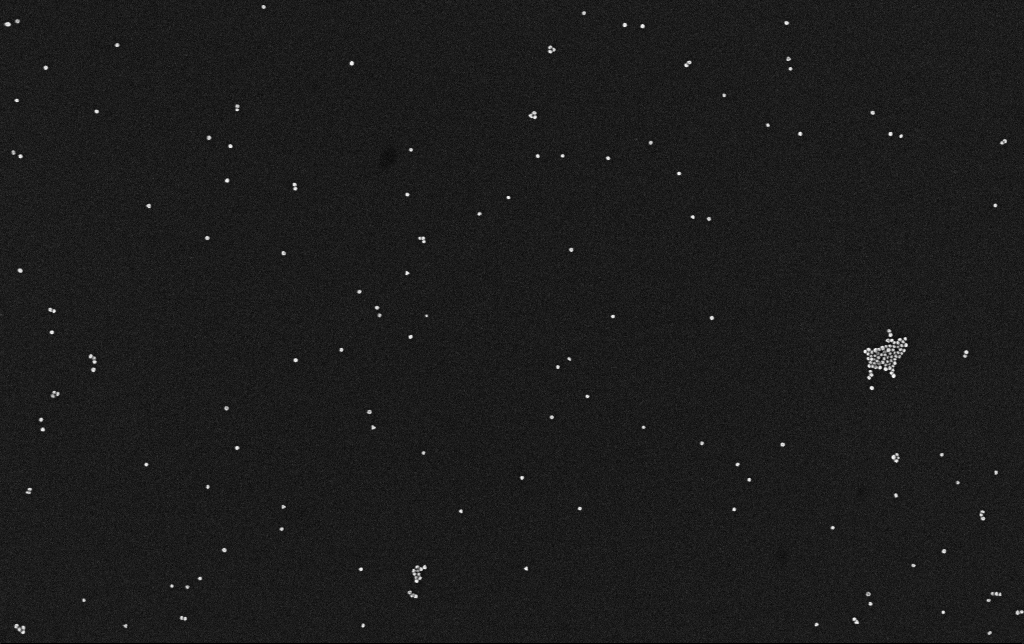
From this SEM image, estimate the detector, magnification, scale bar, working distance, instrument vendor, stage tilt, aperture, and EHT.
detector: InLens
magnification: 100 K X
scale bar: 200 nm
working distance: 3.1 mm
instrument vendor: Zeiss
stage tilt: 0°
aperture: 30 µm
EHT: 10 kV